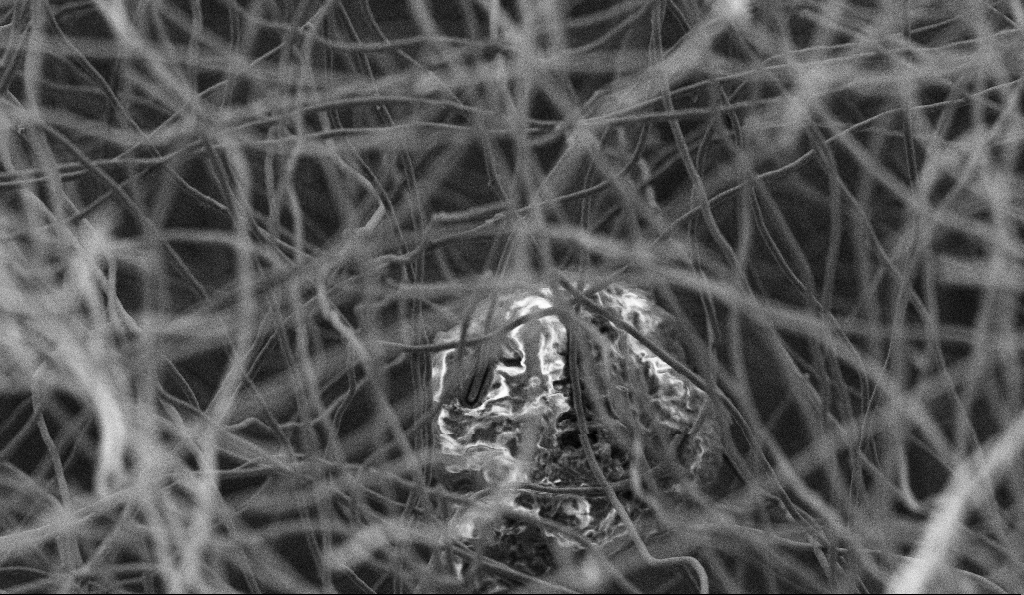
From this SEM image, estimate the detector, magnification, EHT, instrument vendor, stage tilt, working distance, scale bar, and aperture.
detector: SE2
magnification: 5 K X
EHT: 3 kV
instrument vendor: Zeiss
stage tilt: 0°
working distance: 5 mm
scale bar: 10000 nm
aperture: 30 µm